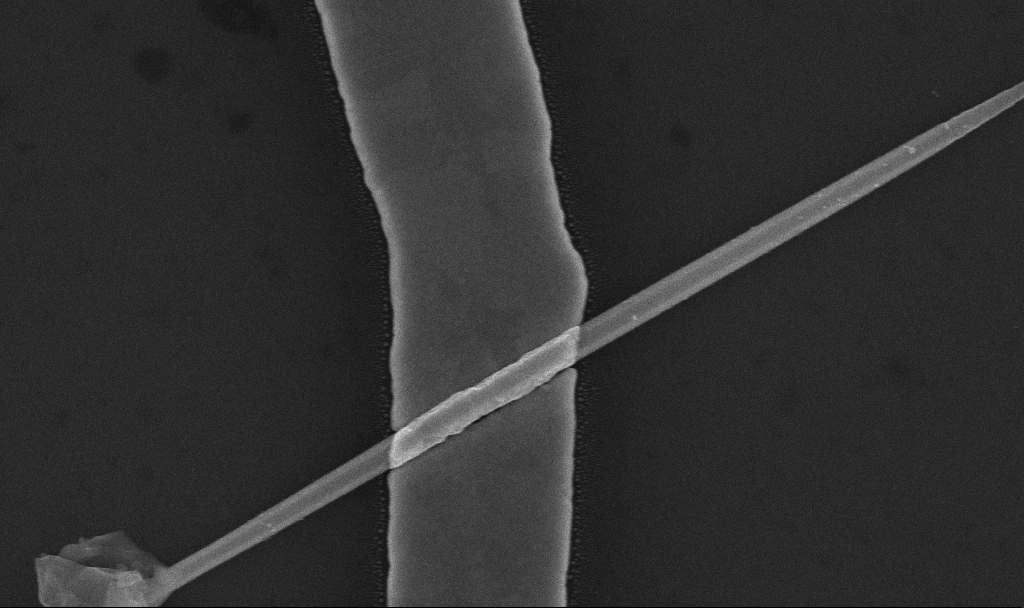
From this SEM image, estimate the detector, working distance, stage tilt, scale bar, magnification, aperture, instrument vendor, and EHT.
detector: InLens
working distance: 7.6 mm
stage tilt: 0°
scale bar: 200 nm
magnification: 77.01 K X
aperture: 30 µm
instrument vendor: Zeiss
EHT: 10 kV